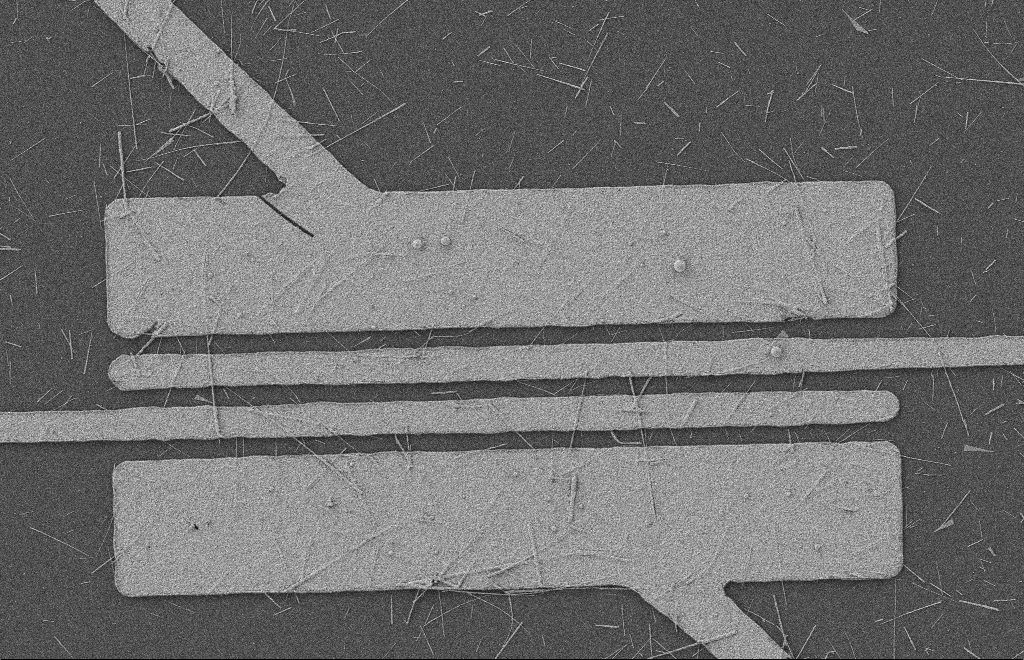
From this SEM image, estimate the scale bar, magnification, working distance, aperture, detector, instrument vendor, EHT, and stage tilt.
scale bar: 2000 nm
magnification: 4.77 K X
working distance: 8 mm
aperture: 20 µm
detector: SE2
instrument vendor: Zeiss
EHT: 2 kV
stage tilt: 0°